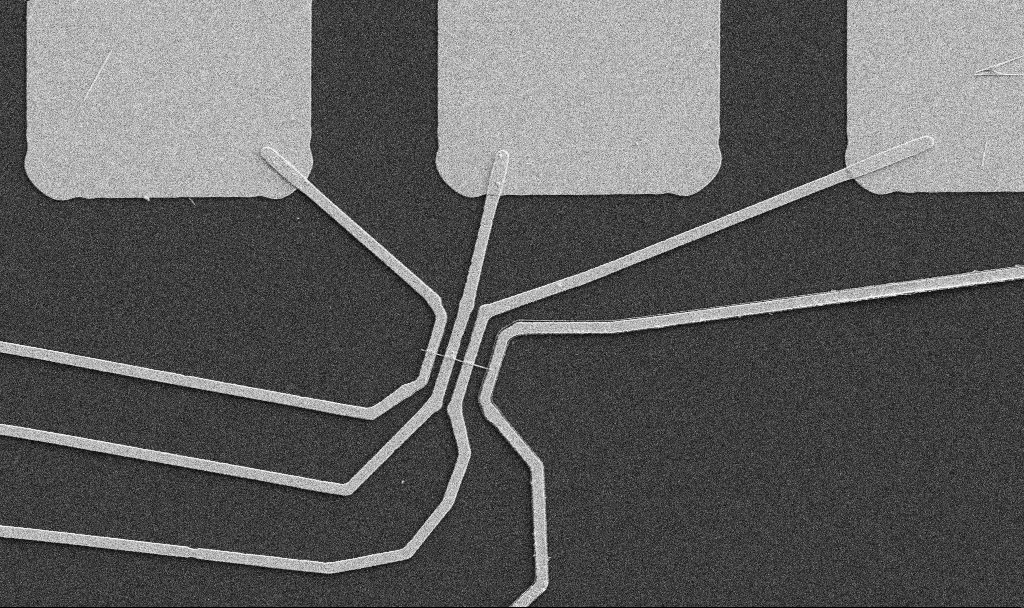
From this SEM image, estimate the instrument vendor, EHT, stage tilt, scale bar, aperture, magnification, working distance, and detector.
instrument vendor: Zeiss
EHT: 5 kV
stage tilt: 0°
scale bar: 10000 nm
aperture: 30 µm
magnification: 5 K X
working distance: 10.7 mm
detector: SE2